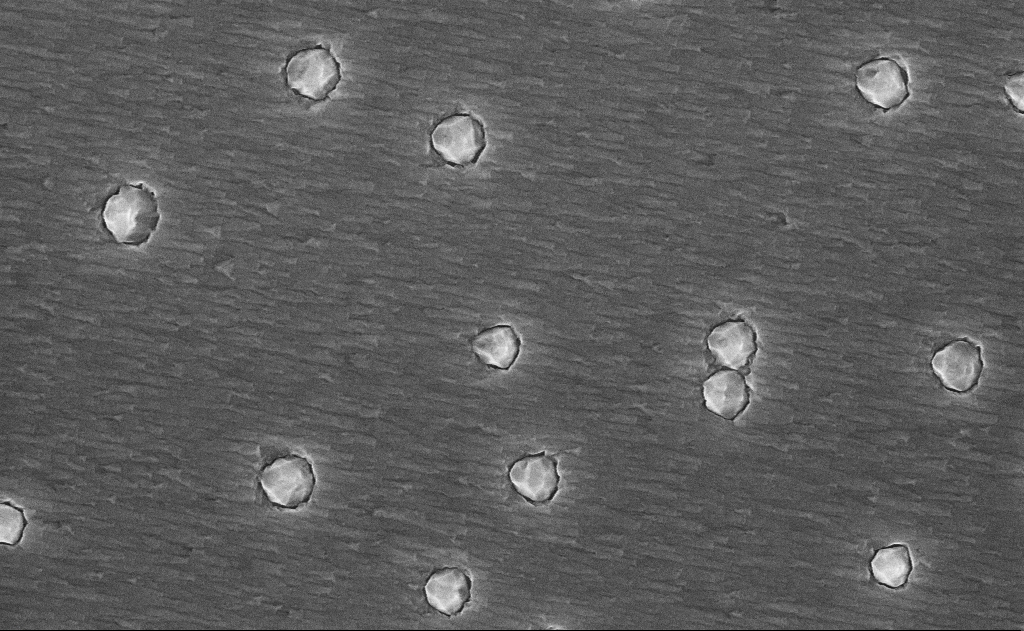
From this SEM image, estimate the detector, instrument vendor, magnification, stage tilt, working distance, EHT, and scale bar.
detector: InLens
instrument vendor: Zeiss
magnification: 40 K X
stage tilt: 0°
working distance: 16 mm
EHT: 10 kV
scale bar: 1000 nm